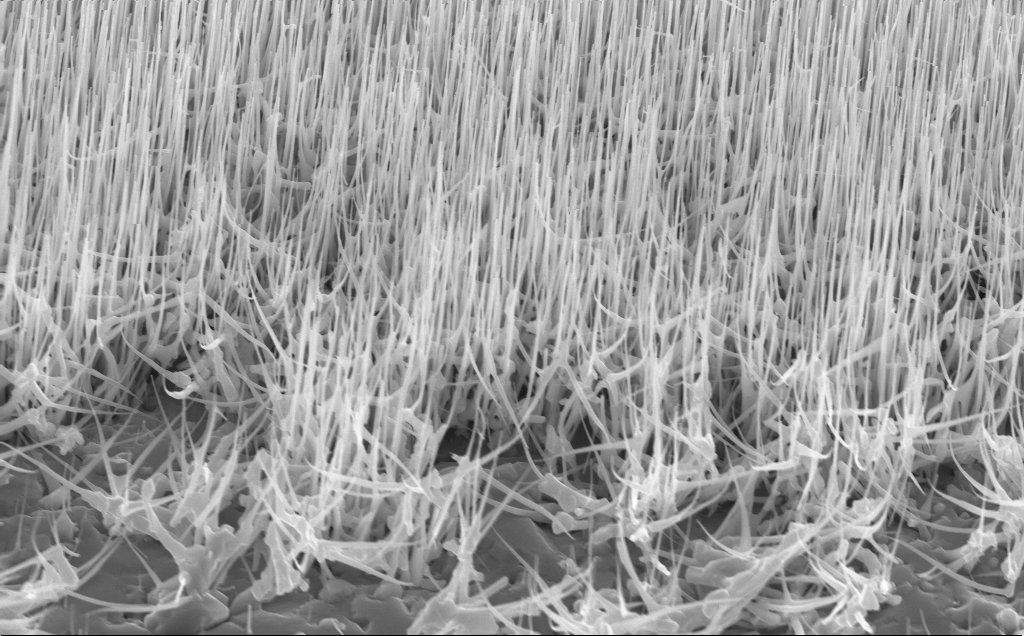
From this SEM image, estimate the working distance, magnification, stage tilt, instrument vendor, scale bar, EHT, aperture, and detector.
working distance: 6 mm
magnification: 19.89 K X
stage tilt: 45°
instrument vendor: Zeiss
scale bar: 2000 nm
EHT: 10 kV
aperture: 30 µm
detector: InLens